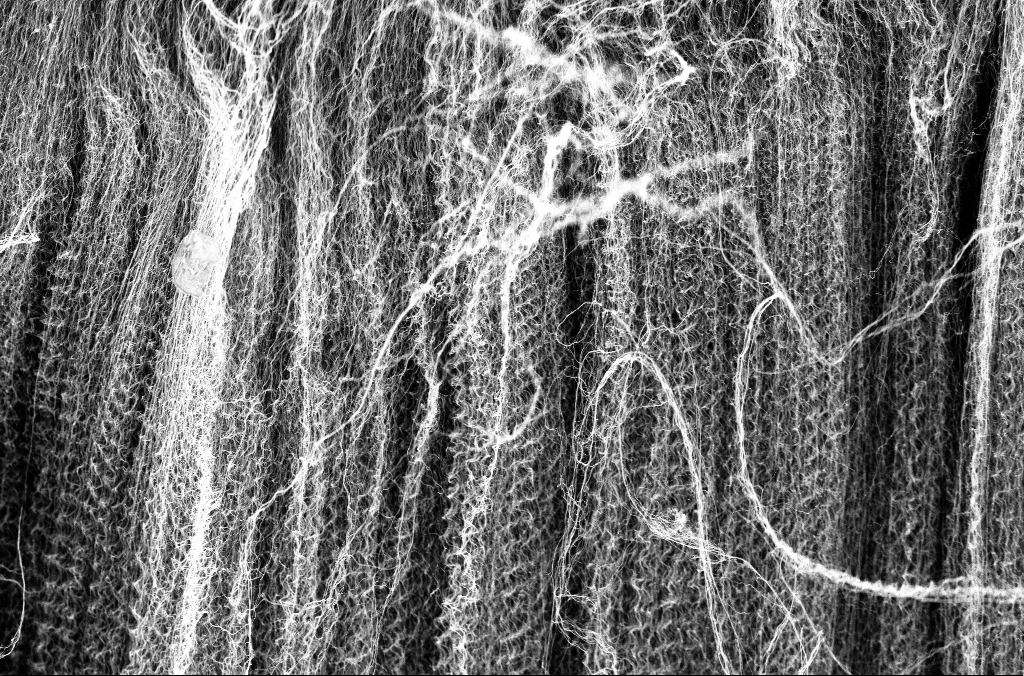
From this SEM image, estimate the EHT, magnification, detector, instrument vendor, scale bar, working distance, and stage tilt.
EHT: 3 kV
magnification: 7.5 K X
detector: InLens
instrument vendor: Zeiss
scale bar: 2000 nm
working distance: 3.3 mm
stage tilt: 45°